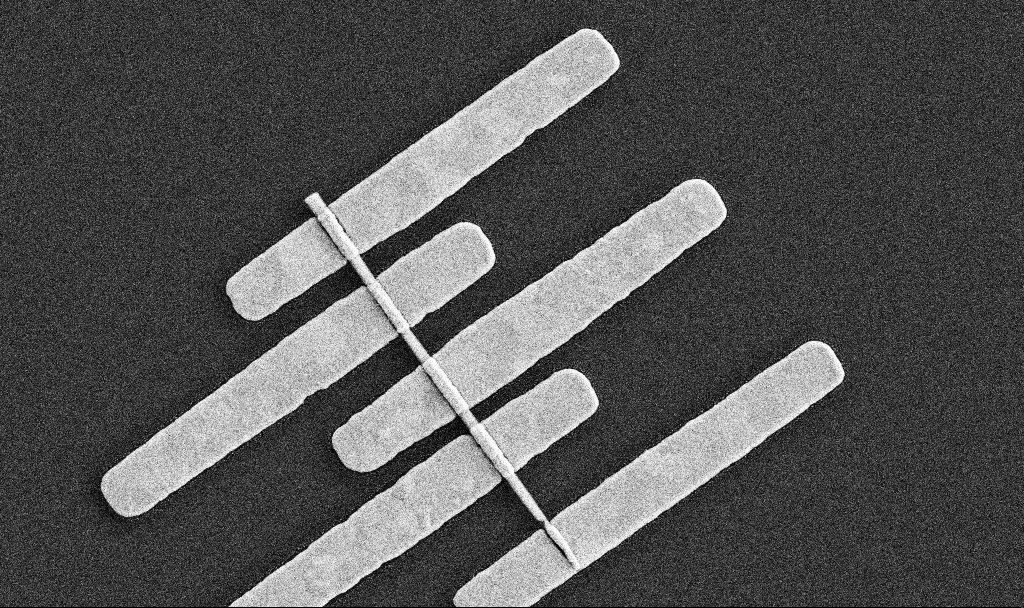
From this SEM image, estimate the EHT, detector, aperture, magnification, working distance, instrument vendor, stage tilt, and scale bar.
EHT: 5 kV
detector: SE2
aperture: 30 µm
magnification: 31.93 K X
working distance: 7.6 mm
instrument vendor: Zeiss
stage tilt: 0°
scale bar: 2000 nm